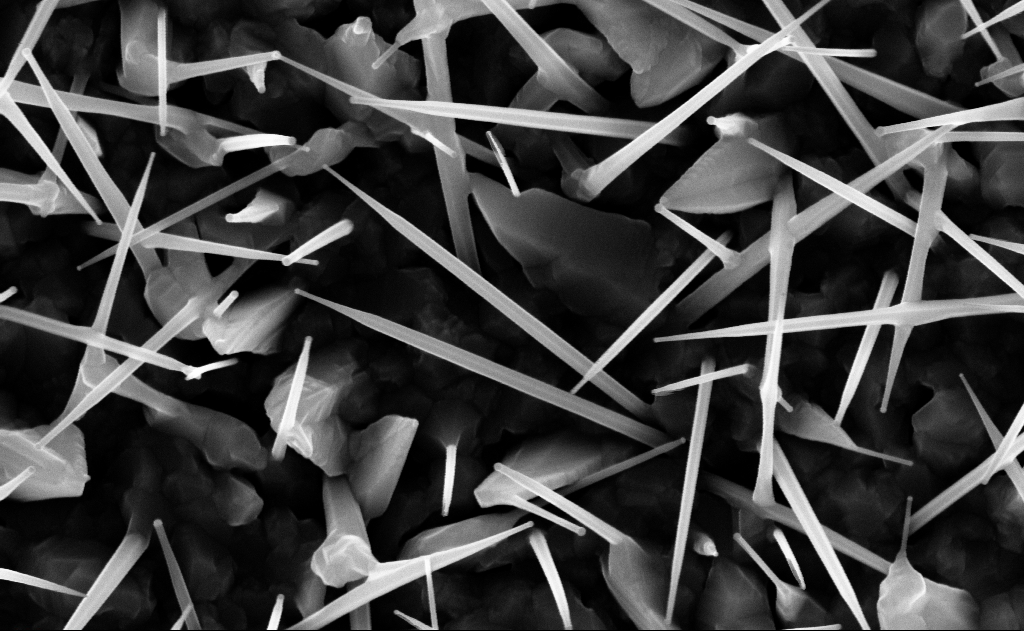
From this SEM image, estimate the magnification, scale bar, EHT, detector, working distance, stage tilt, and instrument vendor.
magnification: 80 K X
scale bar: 200 nm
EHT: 10 kV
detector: InLens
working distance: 9 mm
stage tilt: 0°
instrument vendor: Zeiss